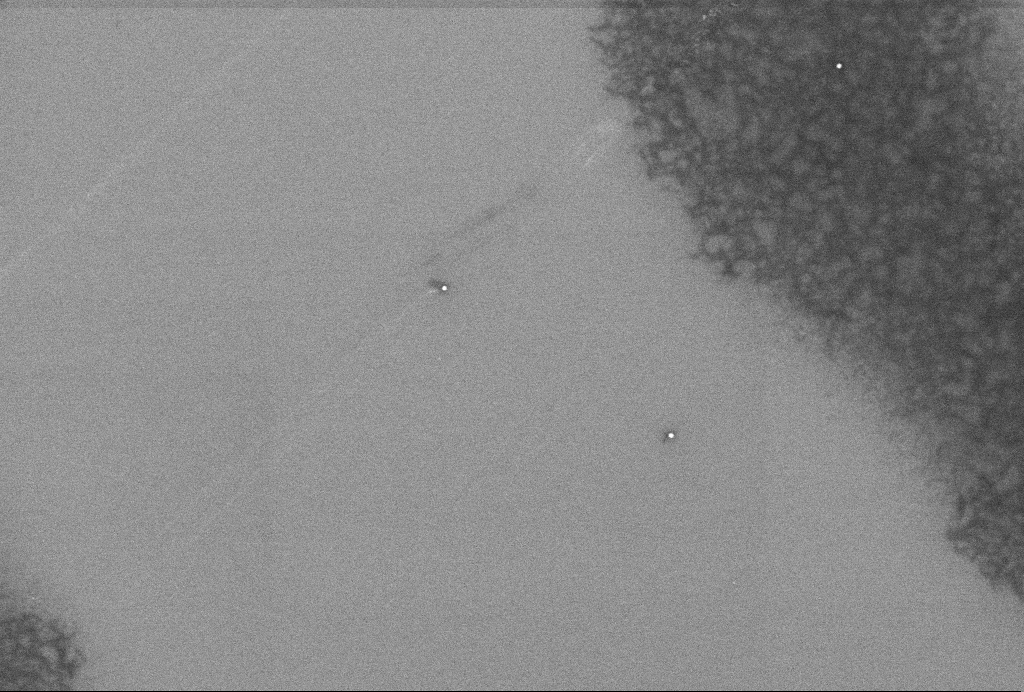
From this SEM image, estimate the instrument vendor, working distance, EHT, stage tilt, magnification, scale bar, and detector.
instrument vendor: Zeiss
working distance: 3.3 mm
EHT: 2 kV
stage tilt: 0°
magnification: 57.52 K X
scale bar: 1000 nm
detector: InLens